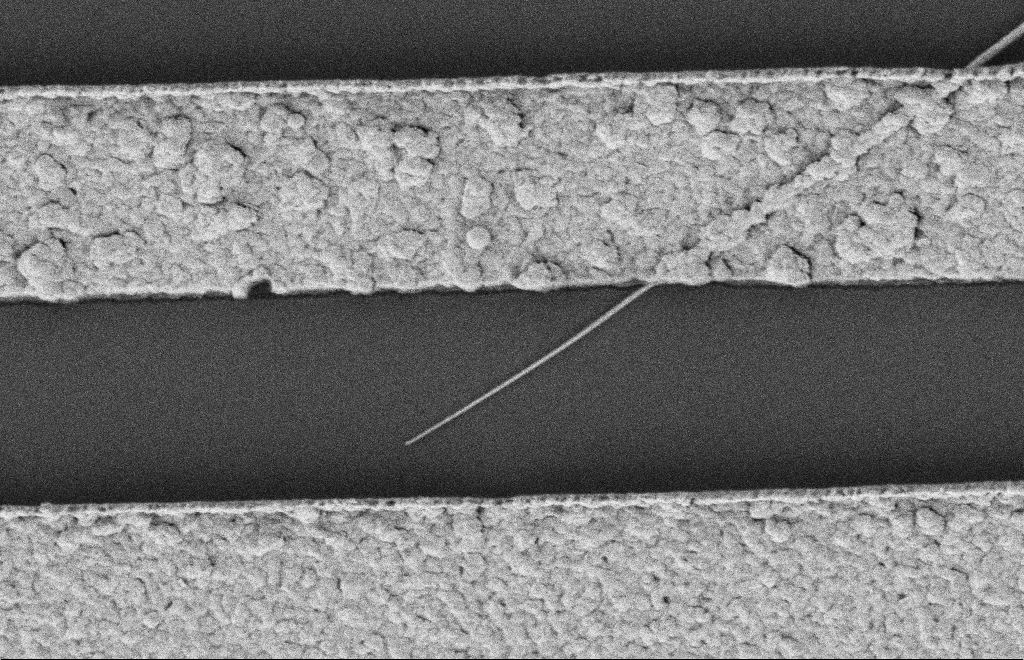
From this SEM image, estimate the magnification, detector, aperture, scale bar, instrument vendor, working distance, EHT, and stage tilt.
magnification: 38.3 K X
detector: SE2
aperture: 20 µm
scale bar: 1000 nm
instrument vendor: Zeiss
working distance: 12 mm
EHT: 2 kV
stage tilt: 0°